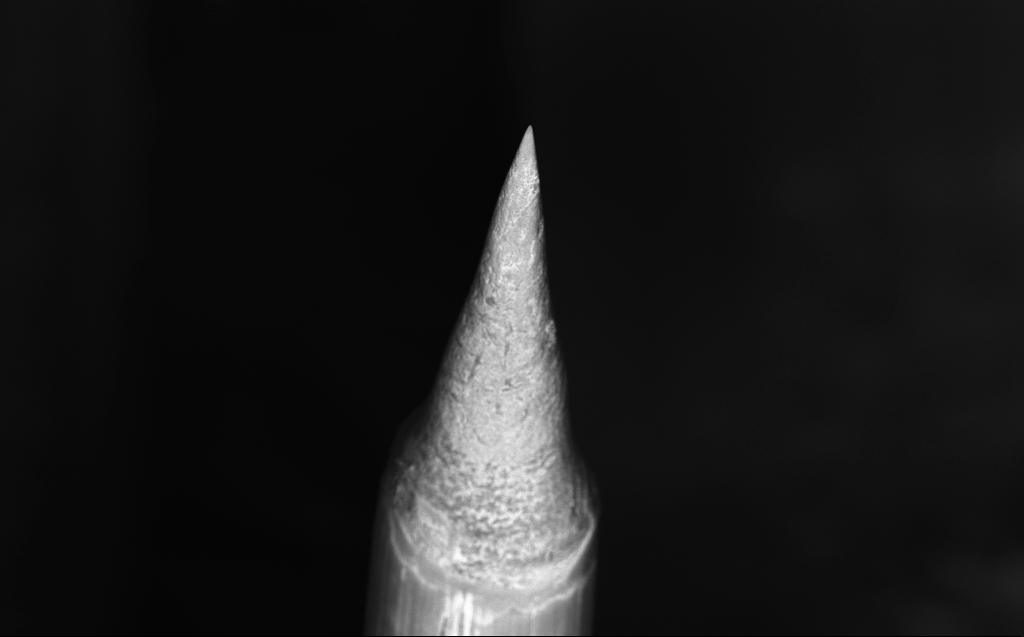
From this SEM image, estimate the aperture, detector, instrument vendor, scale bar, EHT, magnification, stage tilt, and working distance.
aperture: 30 µm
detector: InLens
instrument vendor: Zeiss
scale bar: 100000 nm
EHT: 10 kV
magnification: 0.432 K X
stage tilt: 40°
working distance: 5 mm